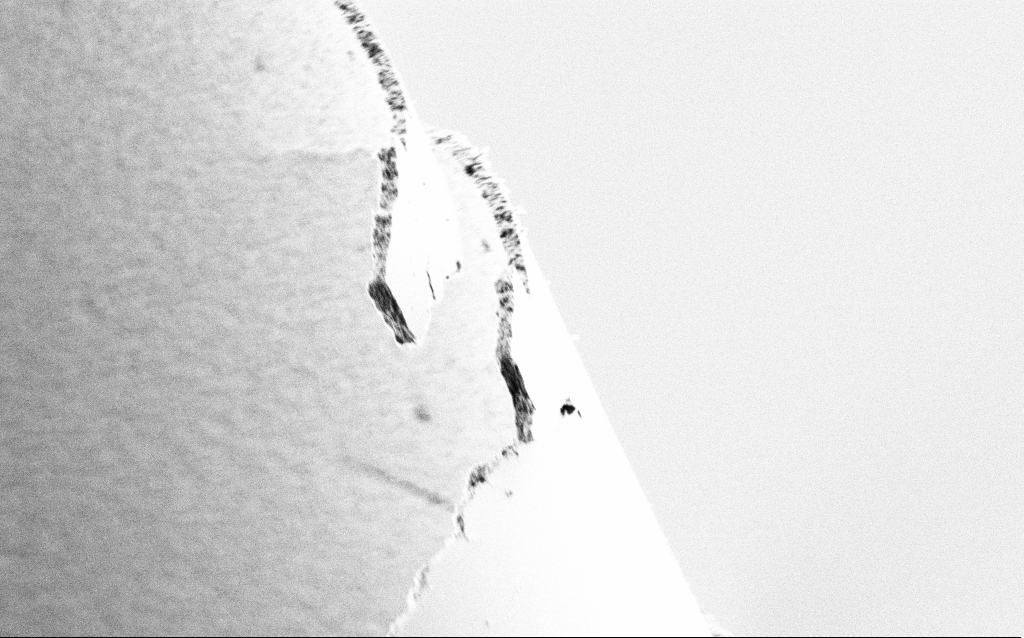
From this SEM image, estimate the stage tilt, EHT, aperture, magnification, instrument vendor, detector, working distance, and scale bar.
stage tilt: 45°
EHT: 1 kV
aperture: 30 µm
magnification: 20 K X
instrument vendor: Zeiss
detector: SE2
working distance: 5 mm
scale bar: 2000 nm